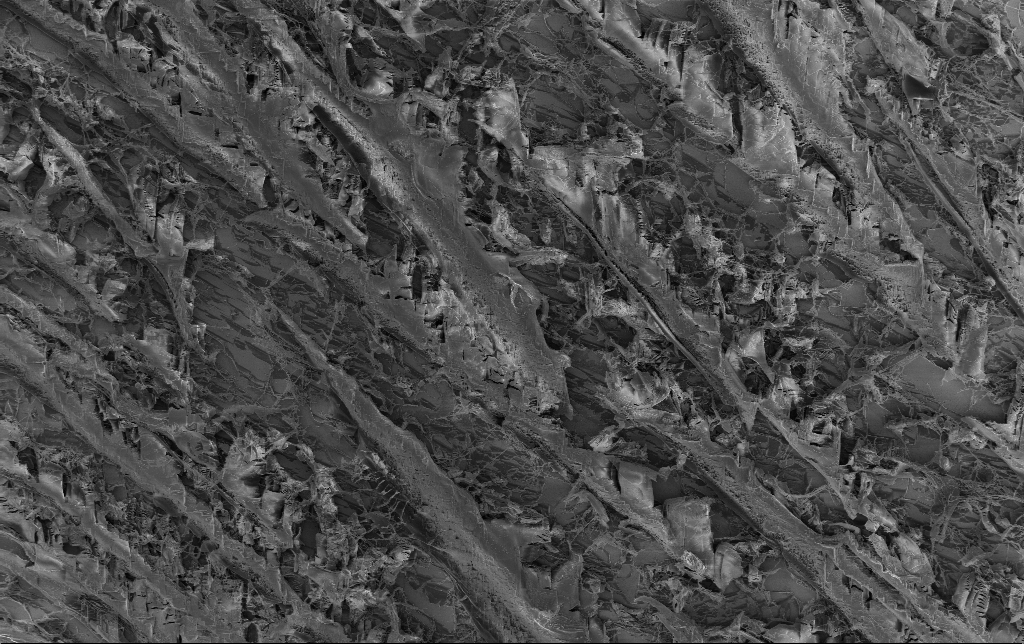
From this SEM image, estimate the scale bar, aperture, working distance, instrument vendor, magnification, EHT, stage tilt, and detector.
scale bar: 20000 nm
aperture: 30 µm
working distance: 3.1 mm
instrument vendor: Zeiss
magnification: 1.07 K X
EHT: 1.5 kV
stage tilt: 0°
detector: InLens